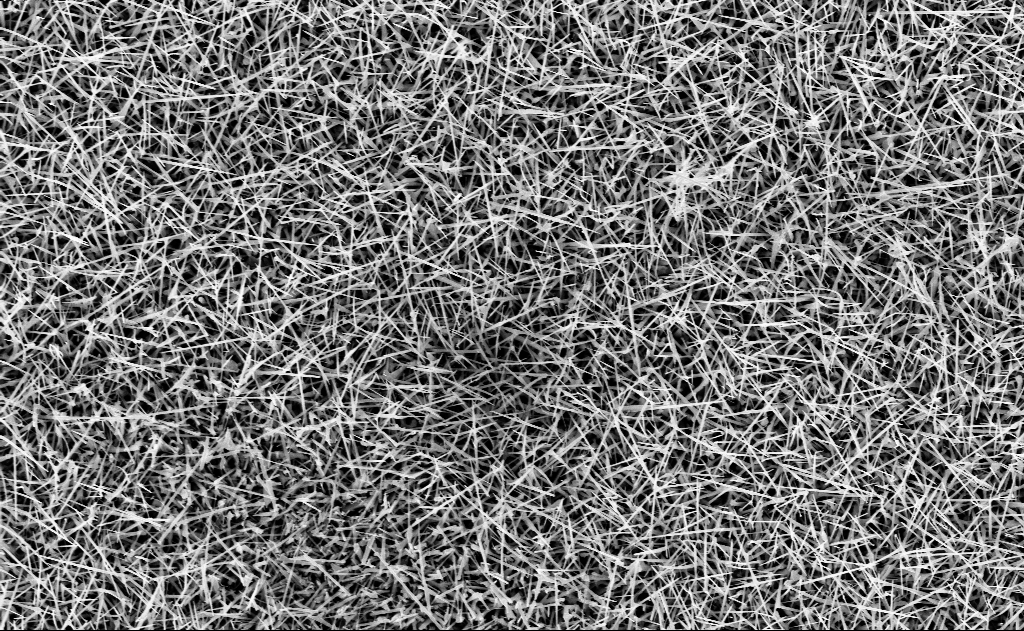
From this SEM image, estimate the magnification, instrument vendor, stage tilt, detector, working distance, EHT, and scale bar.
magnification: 10 K X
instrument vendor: Zeiss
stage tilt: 0°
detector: InLens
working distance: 13 mm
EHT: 10 kV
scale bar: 2000 nm